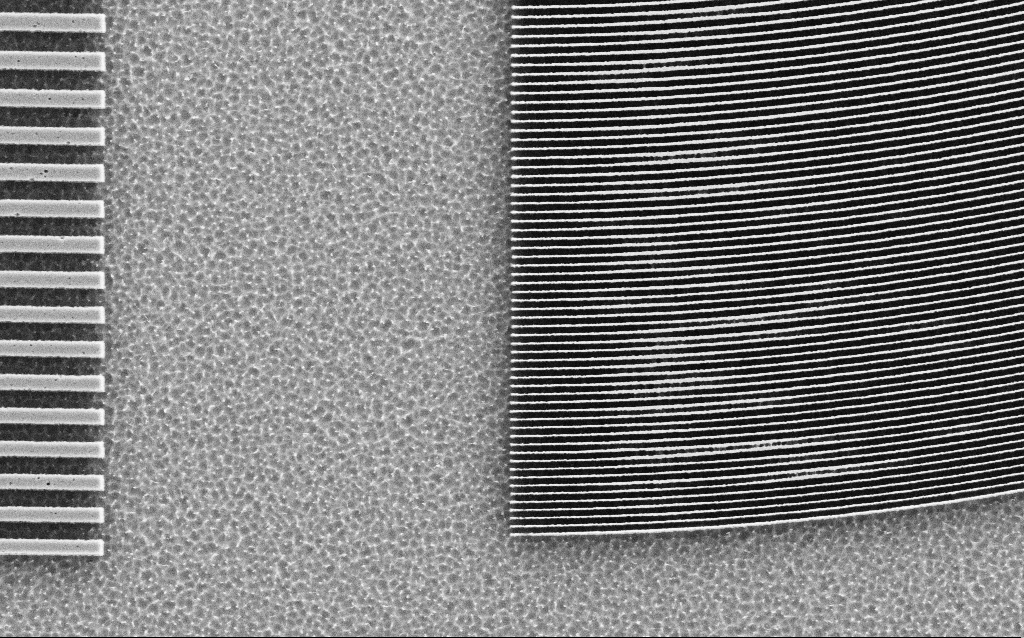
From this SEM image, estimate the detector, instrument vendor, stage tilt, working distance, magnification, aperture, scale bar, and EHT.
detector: SE2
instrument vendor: Zeiss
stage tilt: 0°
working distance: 5 mm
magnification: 14.96 K X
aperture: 30 µm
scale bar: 1000 nm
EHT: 3 kV